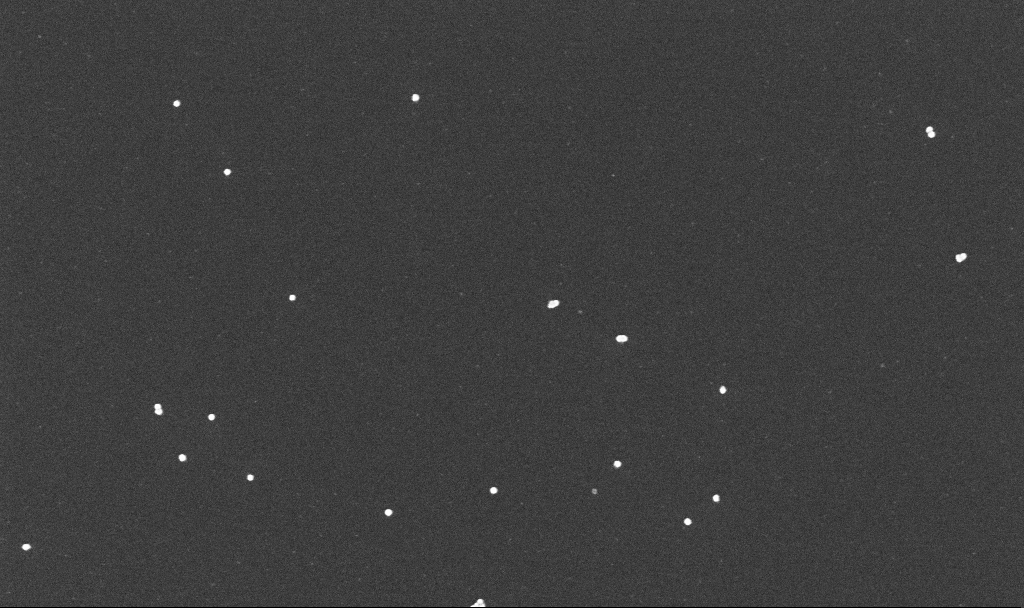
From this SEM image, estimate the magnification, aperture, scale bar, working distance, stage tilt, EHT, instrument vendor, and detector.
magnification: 100 K X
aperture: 30 µm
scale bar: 200 nm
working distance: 3.2 mm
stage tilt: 0°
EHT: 10 kV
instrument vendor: Zeiss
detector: InLens